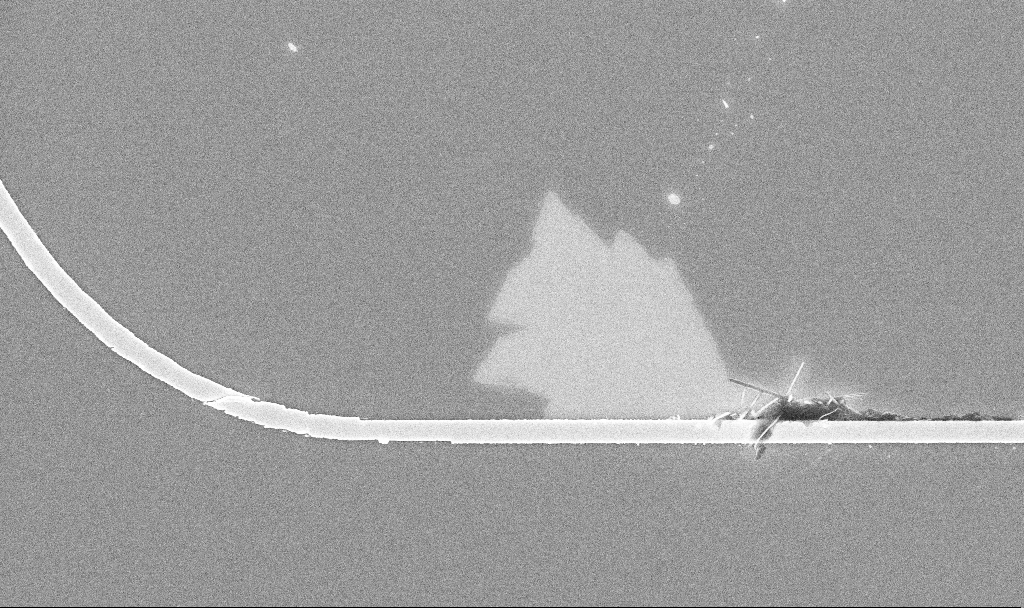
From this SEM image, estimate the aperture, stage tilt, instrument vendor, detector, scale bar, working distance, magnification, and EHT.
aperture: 30 µm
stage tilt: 0°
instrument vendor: Zeiss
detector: InLens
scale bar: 2000 nm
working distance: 10.3 mm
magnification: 16.71 K X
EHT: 5 kV